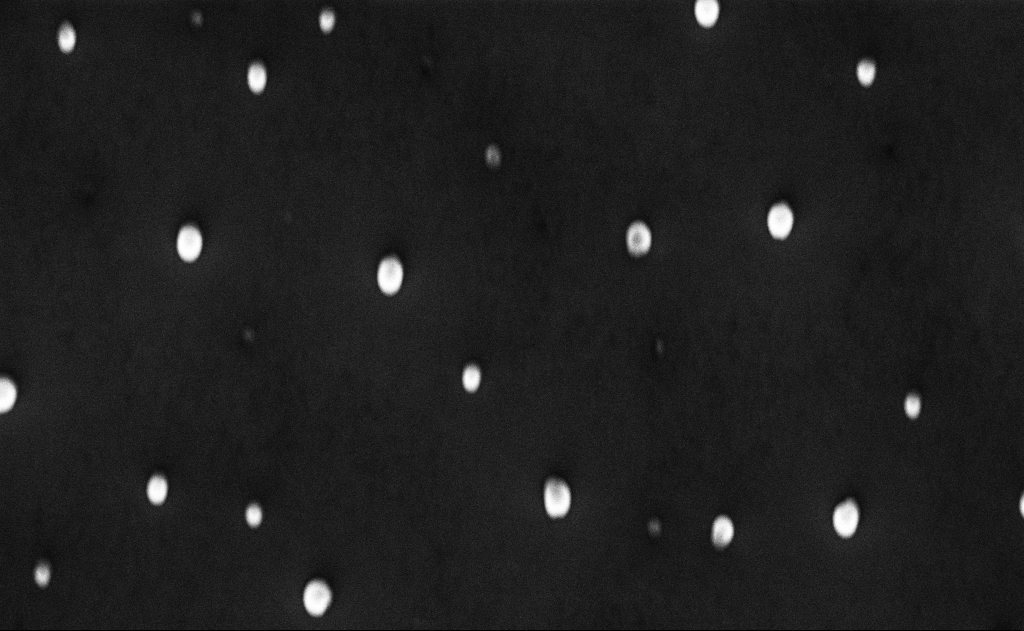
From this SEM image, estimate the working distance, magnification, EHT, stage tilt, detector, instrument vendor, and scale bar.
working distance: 10 mm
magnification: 20 K X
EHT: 10 kV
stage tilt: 0°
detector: InLens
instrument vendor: Zeiss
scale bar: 2000 nm